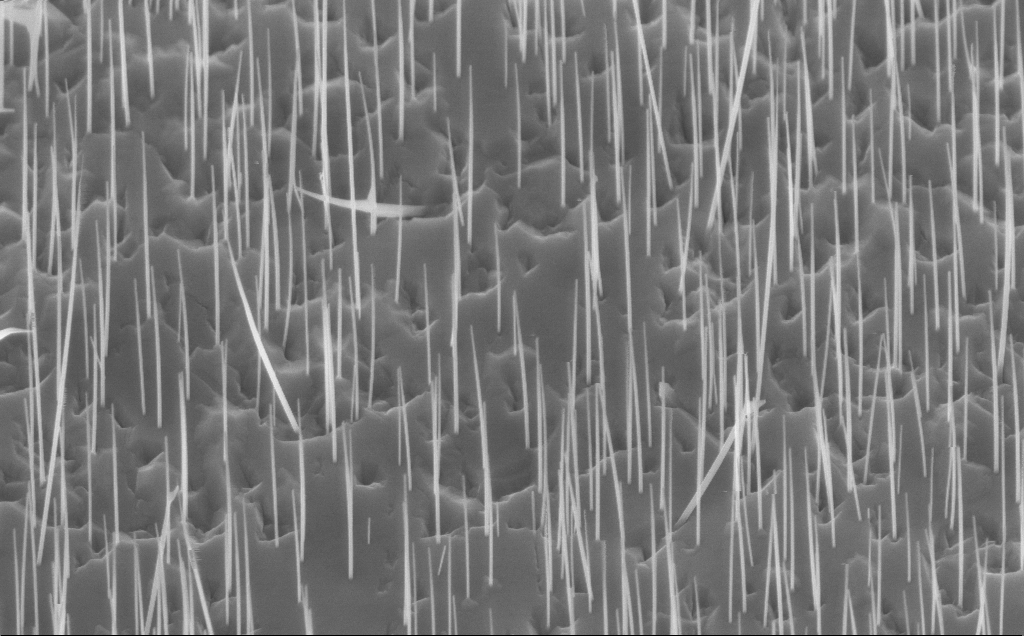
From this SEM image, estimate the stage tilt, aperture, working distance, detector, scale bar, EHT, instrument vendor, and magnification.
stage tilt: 45°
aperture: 30 µm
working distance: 7 mm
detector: InLens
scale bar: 1000 nm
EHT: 10 kV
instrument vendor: Zeiss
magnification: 40 K X